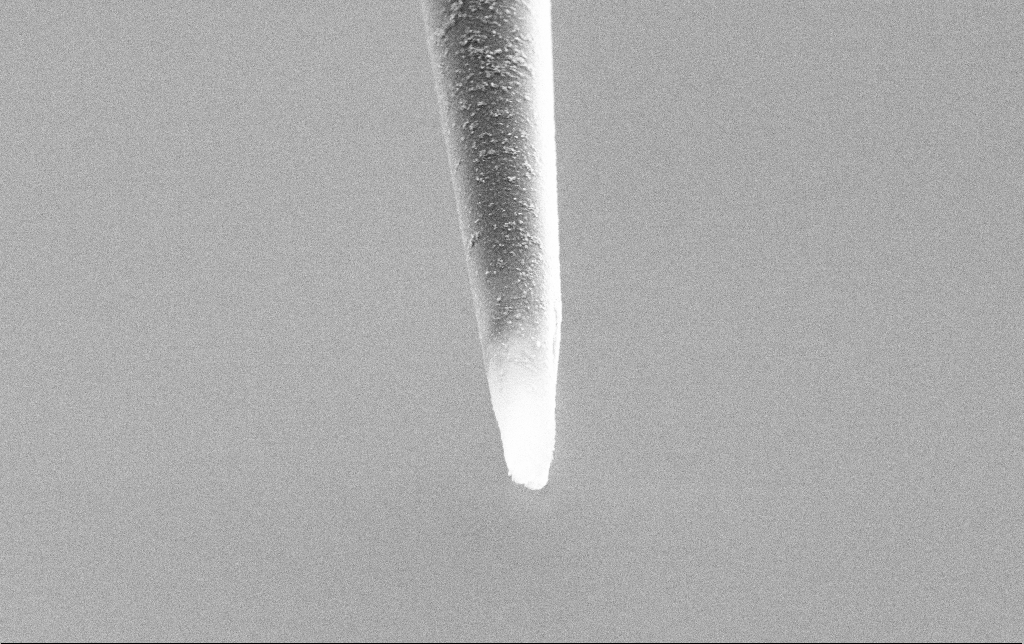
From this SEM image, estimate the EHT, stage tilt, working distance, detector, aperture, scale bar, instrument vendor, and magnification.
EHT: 3 kV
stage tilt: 45°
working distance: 7.5 mm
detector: SE2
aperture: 30 µm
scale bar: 1000 nm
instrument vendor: Zeiss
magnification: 15 K X